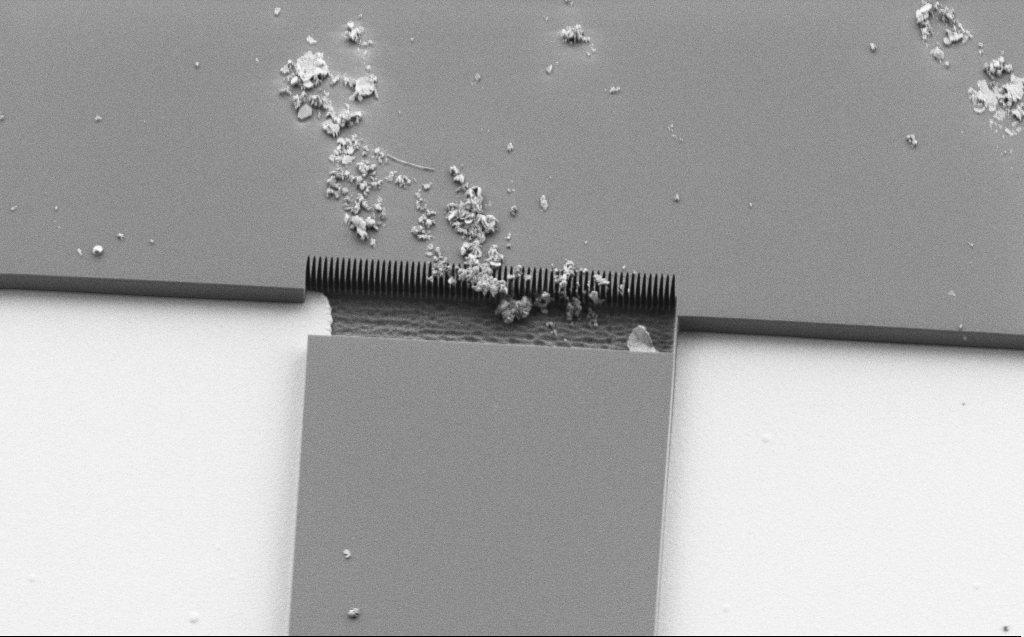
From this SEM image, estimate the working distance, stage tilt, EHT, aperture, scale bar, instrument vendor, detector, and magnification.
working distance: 6 mm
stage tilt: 30°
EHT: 1.1 kV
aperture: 30 µm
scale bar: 10000 nm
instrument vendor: Zeiss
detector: SE2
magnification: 4.27 K X